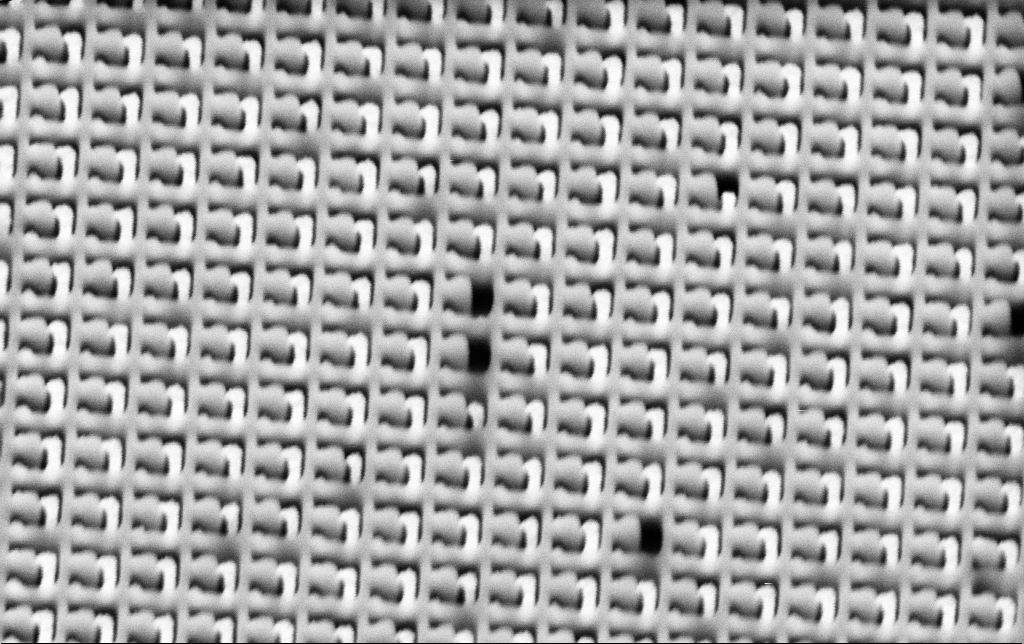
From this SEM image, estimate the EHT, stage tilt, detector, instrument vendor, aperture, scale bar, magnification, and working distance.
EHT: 3 kV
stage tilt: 45°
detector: SE2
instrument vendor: Zeiss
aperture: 30 µm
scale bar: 1000 nm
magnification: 47.58 K X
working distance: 10.4 mm